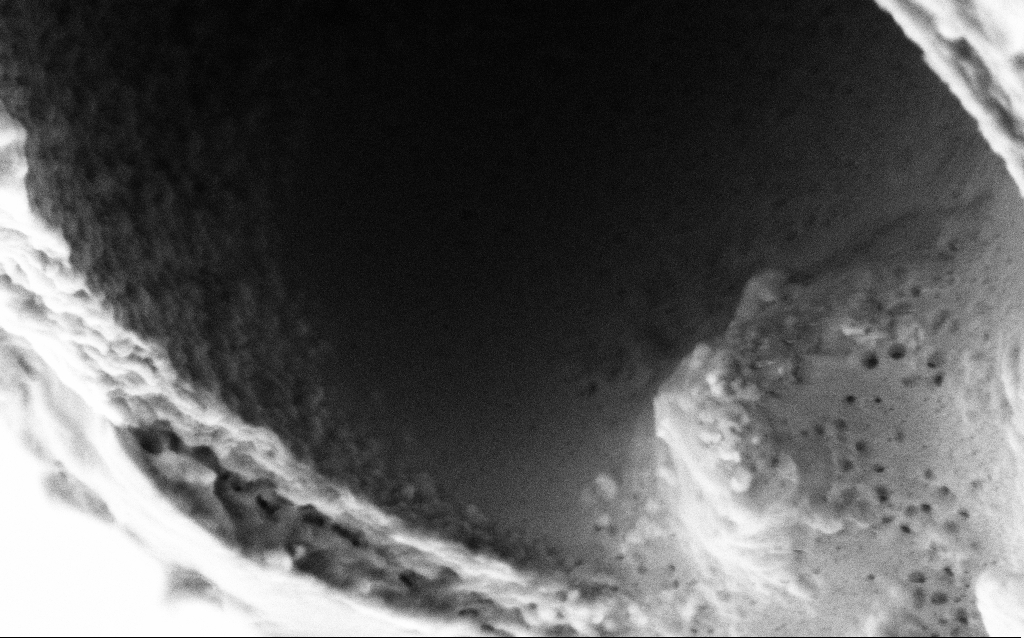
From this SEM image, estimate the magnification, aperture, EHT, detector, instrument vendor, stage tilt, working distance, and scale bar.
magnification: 100 K X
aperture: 30 µm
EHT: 2 kV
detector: SE2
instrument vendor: Zeiss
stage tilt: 45°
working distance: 6 mm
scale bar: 200 nm